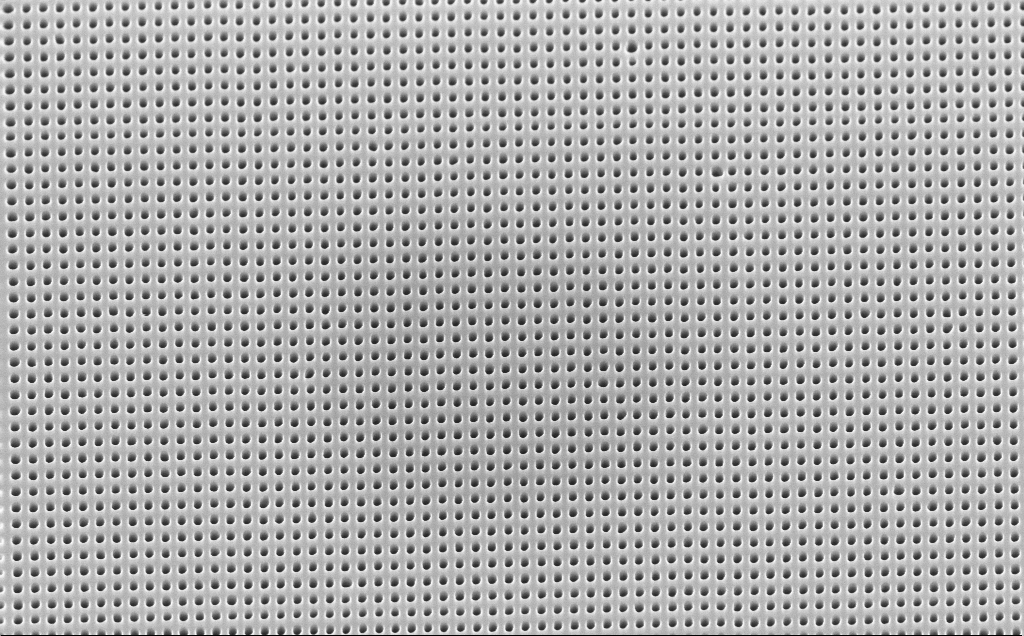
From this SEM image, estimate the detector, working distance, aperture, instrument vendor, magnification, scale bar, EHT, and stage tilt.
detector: InLens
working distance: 4 mm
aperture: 30 µm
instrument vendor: Zeiss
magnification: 40 K X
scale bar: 1000 nm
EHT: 10 kV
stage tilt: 30°